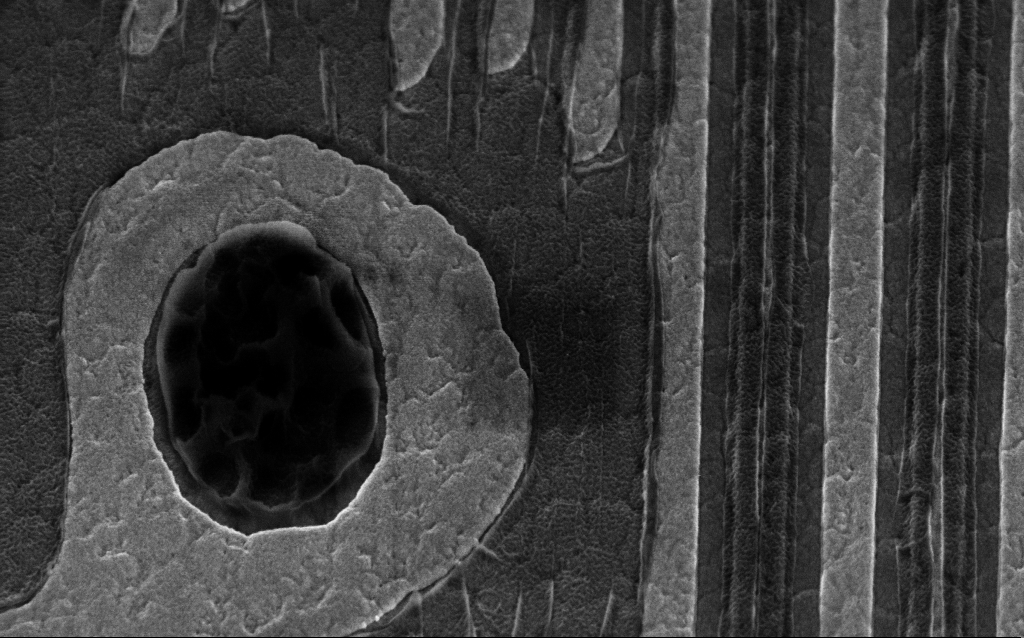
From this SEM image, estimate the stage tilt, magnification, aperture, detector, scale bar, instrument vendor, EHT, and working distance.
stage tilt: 45°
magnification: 65.96 K X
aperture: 30 µm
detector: InLens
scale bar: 1000 nm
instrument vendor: Zeiss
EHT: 3 kV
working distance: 6 mm